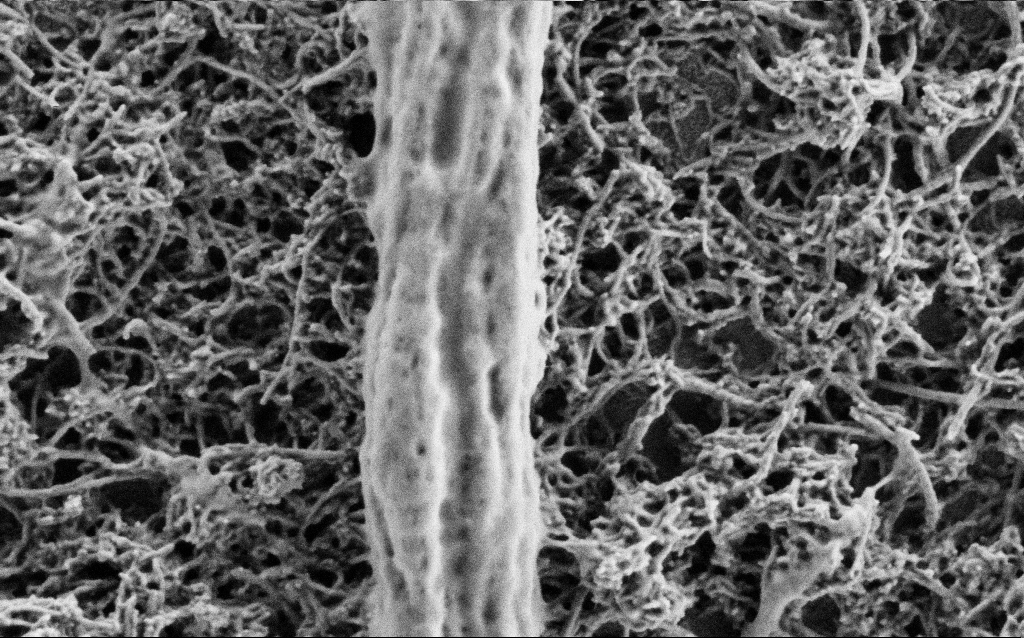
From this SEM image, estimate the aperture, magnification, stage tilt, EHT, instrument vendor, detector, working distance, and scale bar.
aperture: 30 µm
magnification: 75 K X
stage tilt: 0°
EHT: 1 kV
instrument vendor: Zeiss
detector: SE2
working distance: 4 mm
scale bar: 200 nm